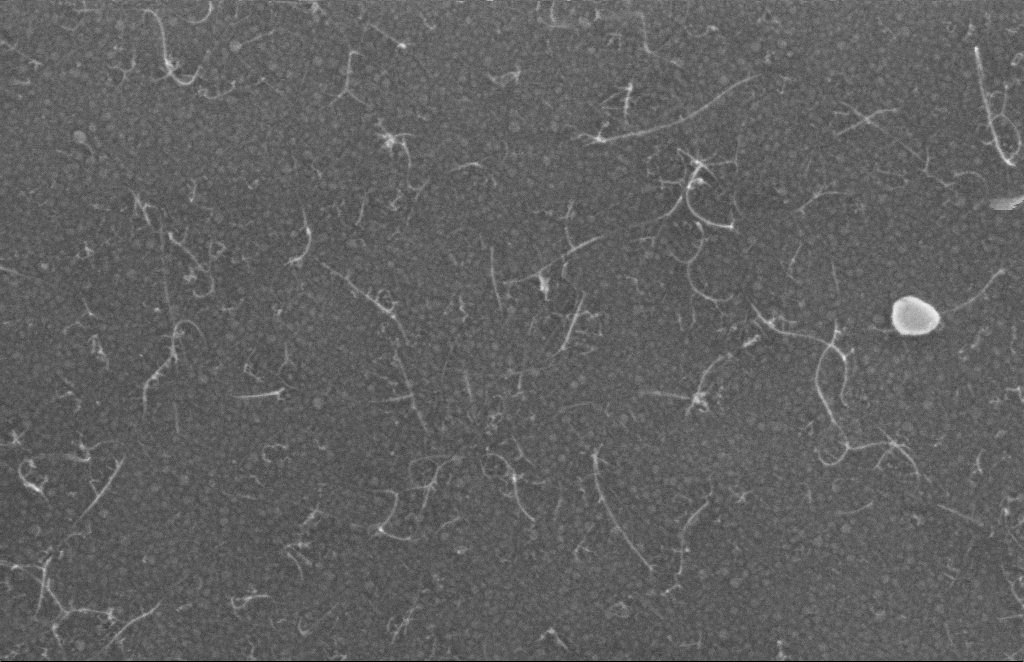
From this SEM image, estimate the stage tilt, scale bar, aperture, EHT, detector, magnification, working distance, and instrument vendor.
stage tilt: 0°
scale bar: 100 nm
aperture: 20 µm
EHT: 5 kV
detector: InLens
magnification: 175.33 K X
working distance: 4 mm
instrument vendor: Zeiss